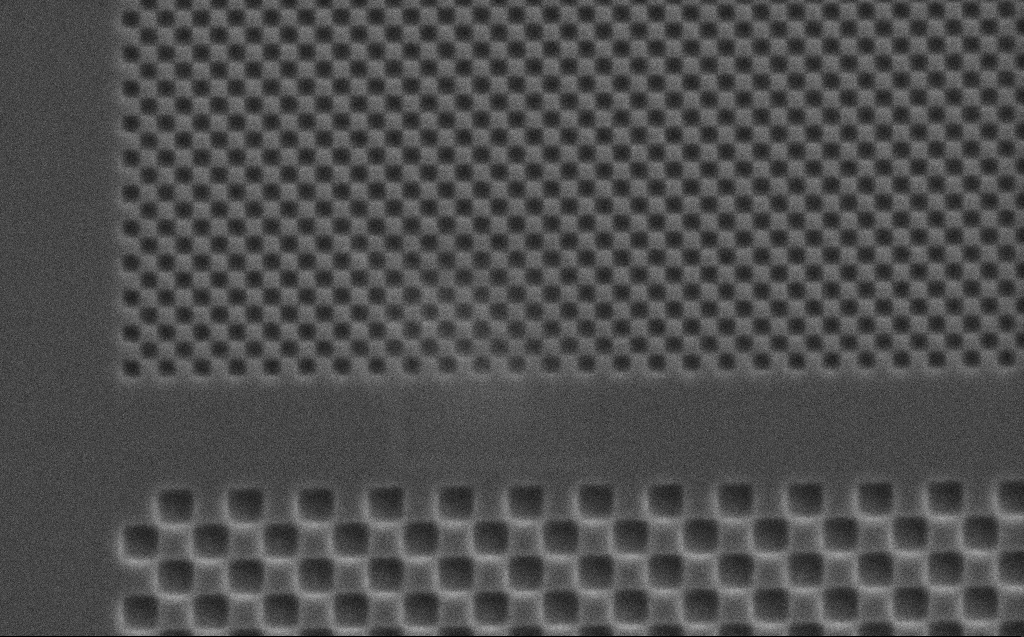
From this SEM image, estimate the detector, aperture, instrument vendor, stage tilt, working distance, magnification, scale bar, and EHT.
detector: SE2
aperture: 30 µm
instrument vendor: Zeiss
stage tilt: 0°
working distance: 5 mm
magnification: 12.99 K X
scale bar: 1000 nm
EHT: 5 kV